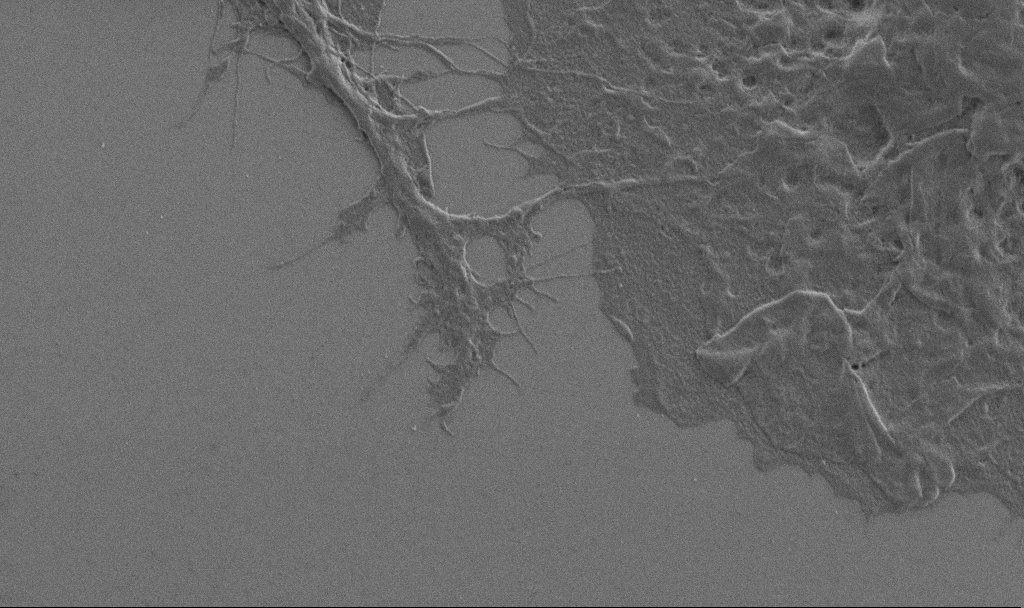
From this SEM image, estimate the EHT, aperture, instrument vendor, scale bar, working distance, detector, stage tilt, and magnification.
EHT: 1 kV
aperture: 30 µm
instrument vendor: Zeiss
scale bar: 2000 nm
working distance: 6.9 mm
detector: SE2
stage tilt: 0°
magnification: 10 K X